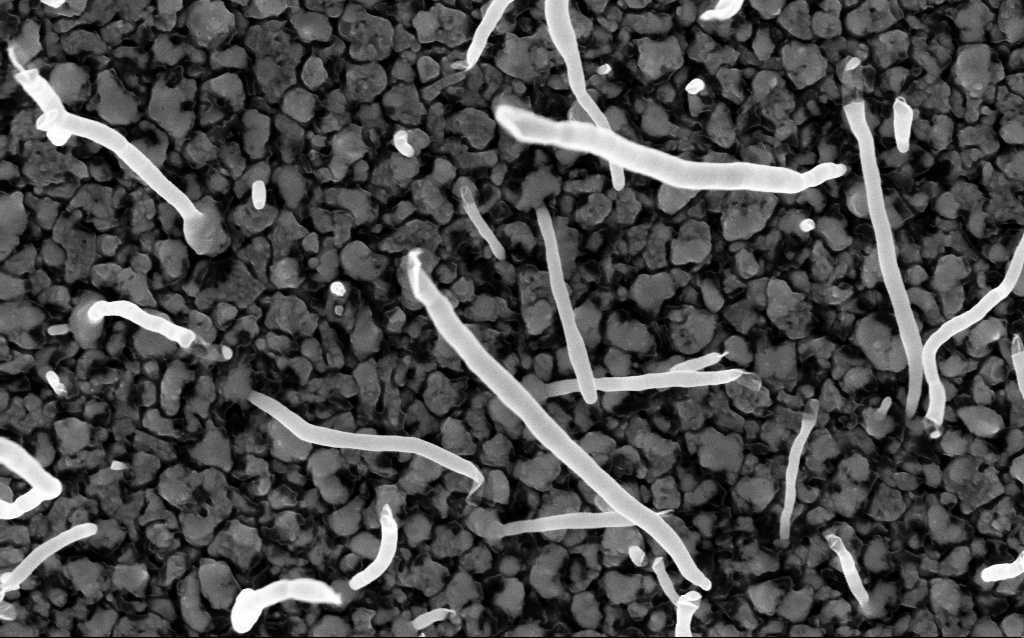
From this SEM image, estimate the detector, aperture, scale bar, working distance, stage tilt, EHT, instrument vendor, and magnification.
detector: InLens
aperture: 30 µm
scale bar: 200 nm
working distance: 2.2 mm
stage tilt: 0°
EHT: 5 kV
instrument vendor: Zeiss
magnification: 100 K X